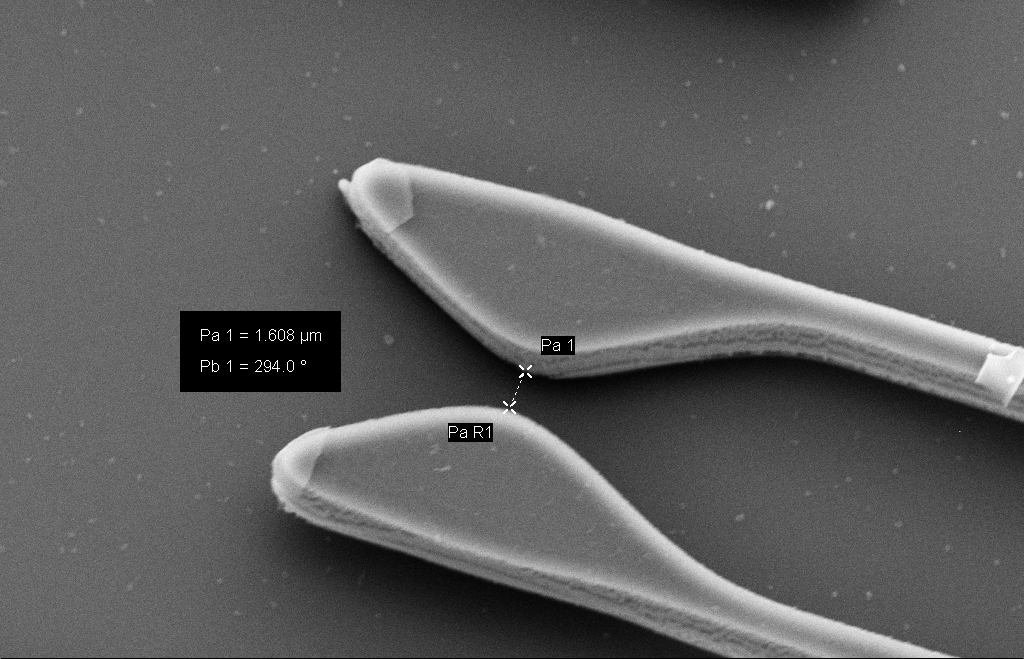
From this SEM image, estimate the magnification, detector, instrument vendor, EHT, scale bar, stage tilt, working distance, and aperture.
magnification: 9 K X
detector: SE2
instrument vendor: Zeiss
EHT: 10 kV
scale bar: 2000 nm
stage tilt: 15.3°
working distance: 11 mm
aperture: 30 µm